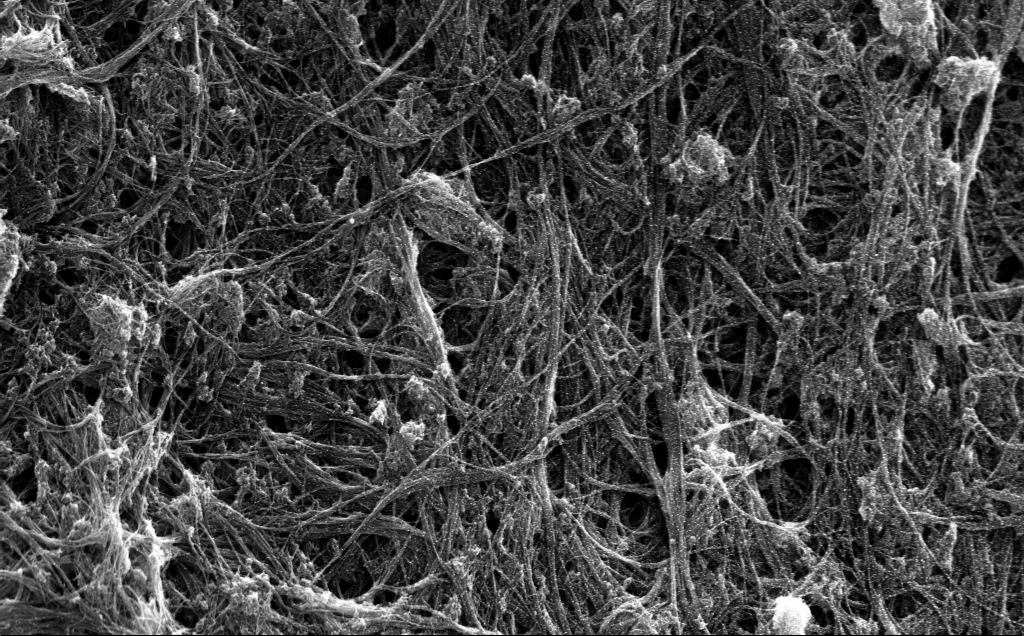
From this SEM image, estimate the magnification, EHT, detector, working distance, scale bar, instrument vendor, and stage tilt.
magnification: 46.4 K X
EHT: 5 kV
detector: InLens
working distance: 4 mm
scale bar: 1000 nm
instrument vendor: Zeiss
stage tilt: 0°